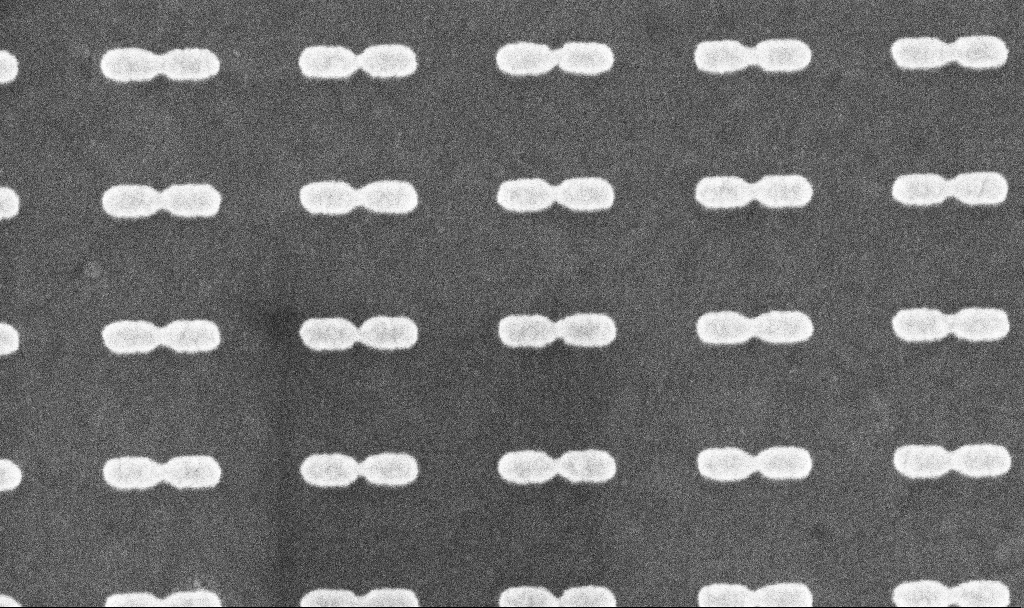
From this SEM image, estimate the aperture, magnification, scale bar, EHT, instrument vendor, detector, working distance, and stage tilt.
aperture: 30 µm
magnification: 167.27 K X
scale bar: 200 nm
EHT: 5 kV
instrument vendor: Zeiss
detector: InLens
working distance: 4.1 mm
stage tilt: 0°